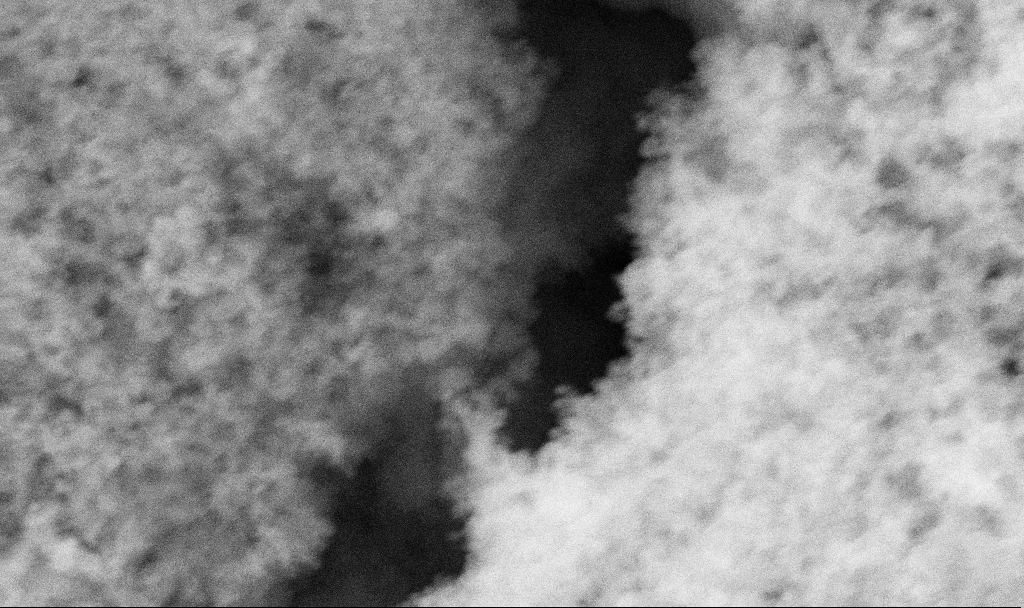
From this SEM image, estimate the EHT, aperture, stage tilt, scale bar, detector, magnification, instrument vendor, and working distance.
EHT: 10 kV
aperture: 30 µm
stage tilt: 0°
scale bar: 200 nm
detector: SE2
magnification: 100.15 K X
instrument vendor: Zeiss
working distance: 2.7 mm